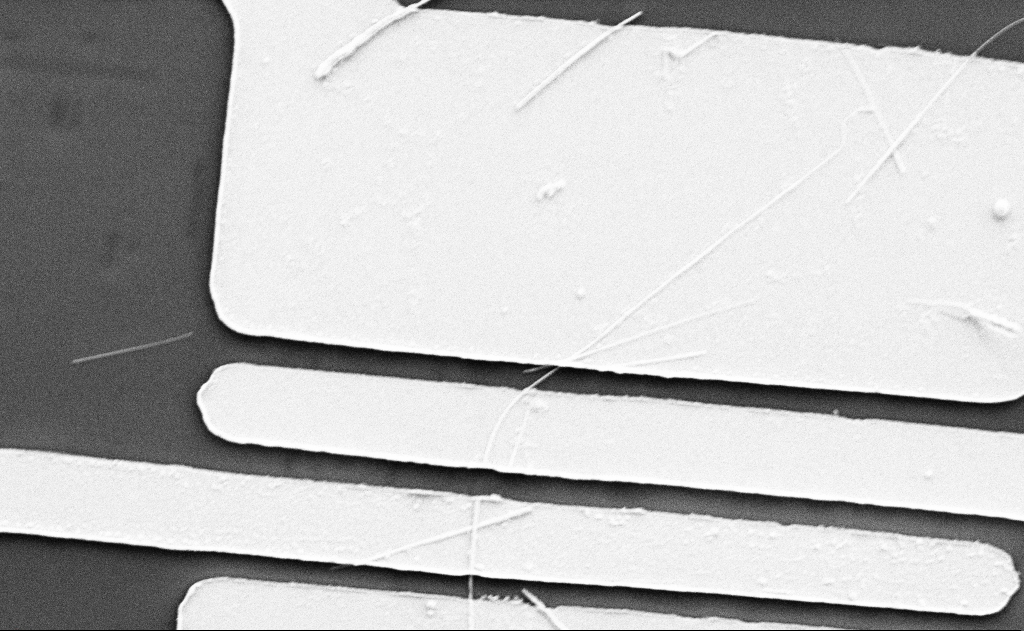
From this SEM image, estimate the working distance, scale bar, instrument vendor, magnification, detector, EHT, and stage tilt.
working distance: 15 mm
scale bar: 2000 nm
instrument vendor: Zeiss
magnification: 10 K X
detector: SE2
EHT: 5 kV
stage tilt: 0°